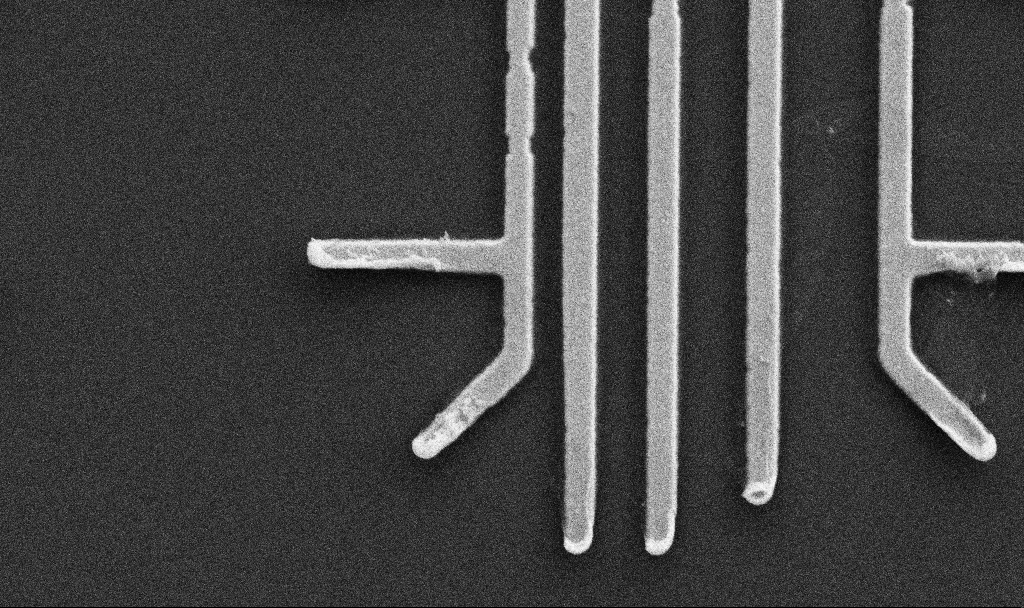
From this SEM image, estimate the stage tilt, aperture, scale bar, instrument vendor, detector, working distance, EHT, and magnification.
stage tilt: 0°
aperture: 30 µm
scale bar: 1000 nm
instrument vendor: Zeiss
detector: SE2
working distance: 10.7 mm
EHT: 5 kV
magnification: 36.63 K X